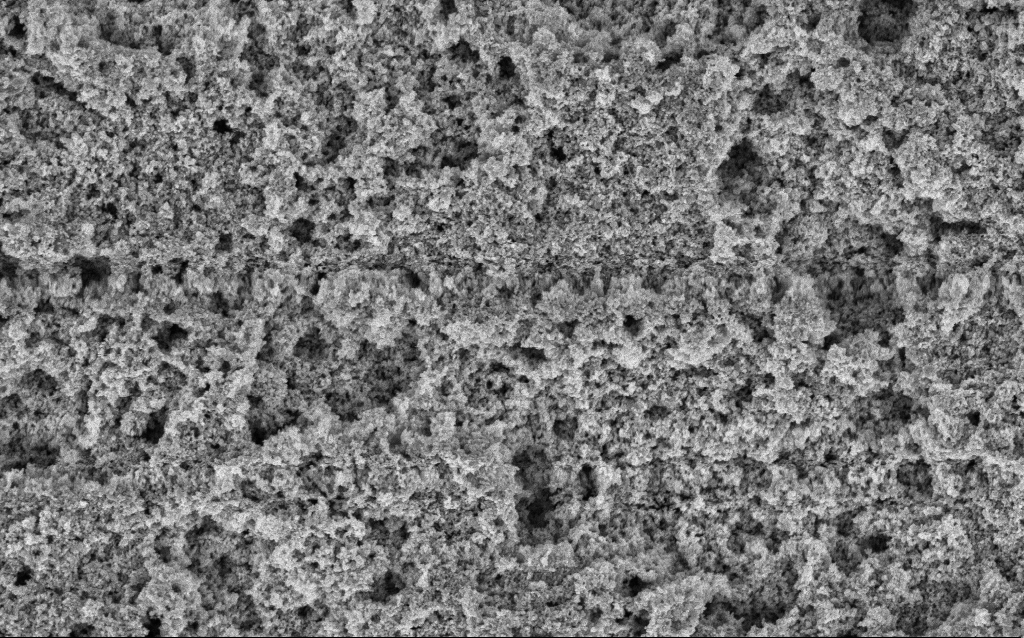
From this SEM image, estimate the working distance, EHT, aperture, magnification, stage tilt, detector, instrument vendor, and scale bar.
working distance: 10 mm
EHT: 3 kV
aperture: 30 µm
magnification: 37.88 K X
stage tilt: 0°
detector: InLens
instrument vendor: Zeiss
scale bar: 1000 nm